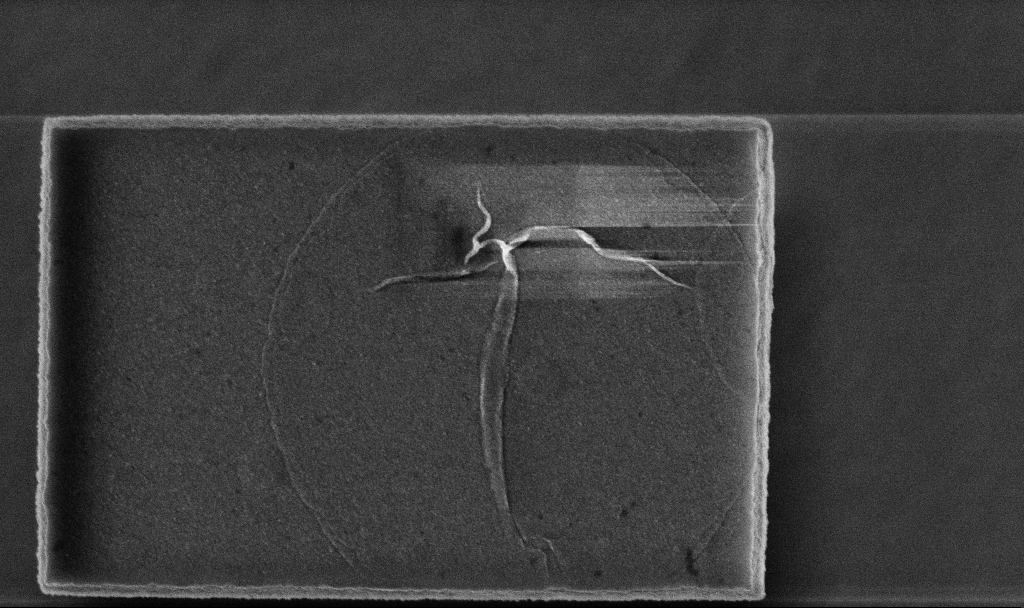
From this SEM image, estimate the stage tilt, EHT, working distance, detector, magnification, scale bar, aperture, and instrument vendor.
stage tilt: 0°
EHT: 5 kV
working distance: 3.2 mm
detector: InLens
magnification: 58.69 K X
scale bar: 1000 nm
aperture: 30 µm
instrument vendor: Zeiss